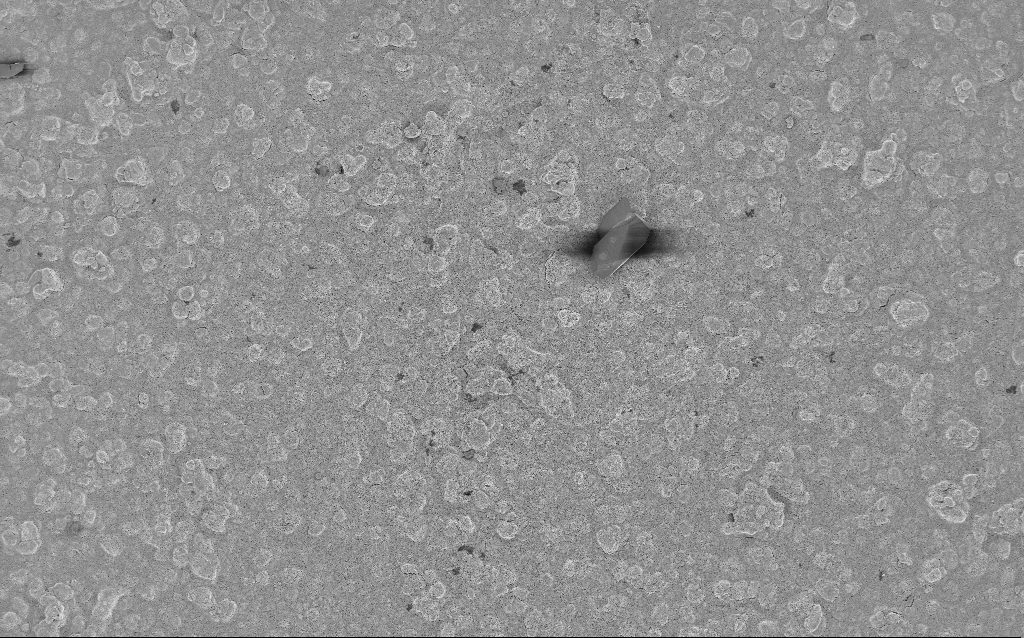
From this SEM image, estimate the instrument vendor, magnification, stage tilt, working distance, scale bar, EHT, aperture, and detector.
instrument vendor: Zeiss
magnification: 0.77 K X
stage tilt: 0°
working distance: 7.6 mm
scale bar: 20000 nm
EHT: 3 kV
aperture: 30 µm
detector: InLens